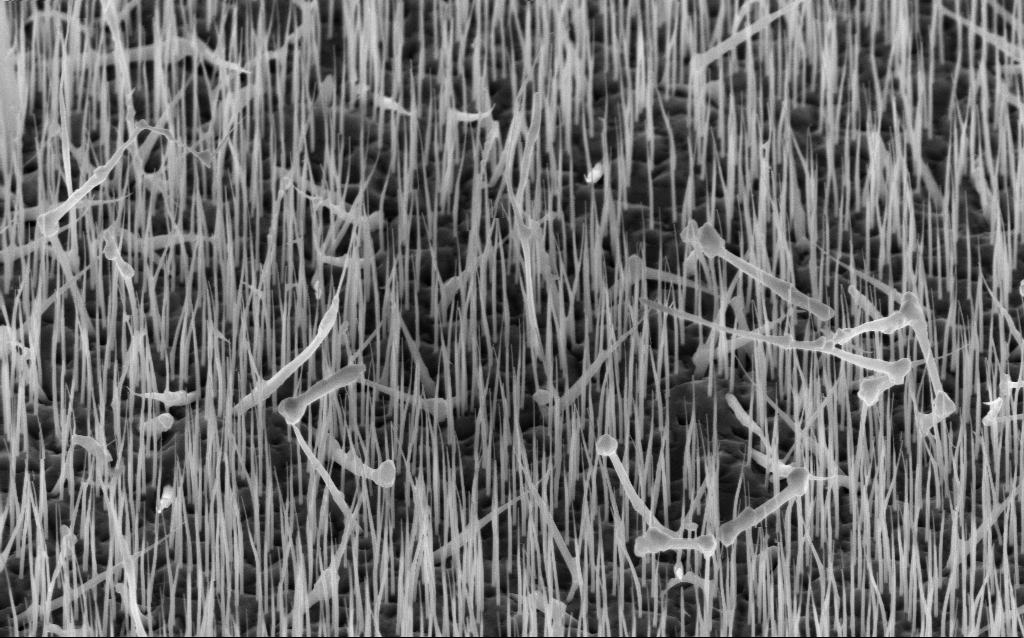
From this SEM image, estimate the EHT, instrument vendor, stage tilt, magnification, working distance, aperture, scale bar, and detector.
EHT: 10 kV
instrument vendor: Zeiss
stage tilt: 45°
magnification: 20 K X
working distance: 6 mm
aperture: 30 µm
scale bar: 1000 nm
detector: InLens